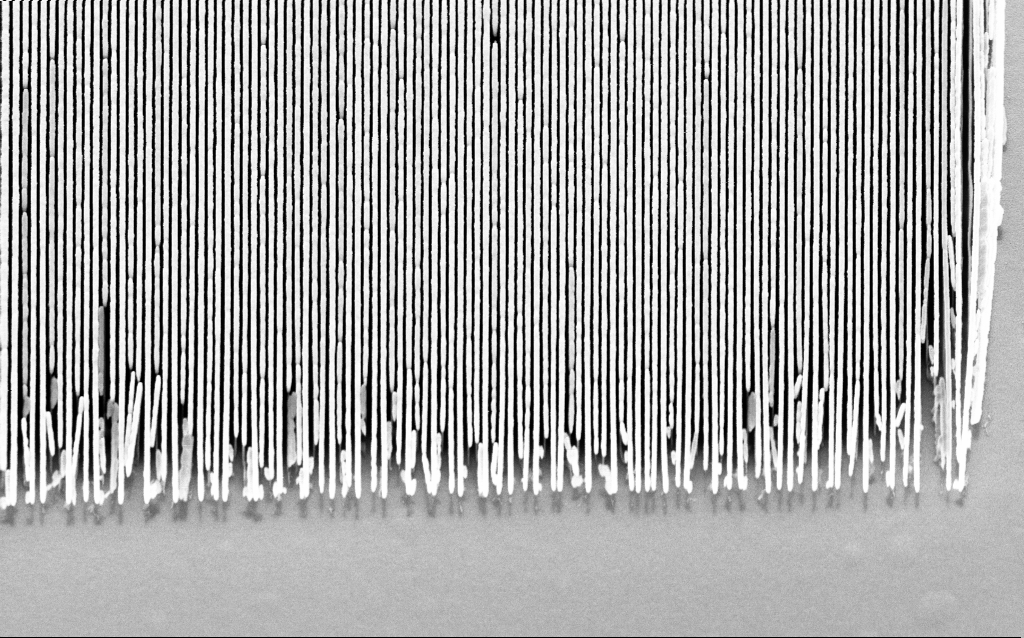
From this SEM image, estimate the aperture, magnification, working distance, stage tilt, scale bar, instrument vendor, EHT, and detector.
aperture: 30 µm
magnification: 31.63 K X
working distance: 4.1 mm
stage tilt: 30°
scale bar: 2000 nm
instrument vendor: Zeiss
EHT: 5 kV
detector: InLens